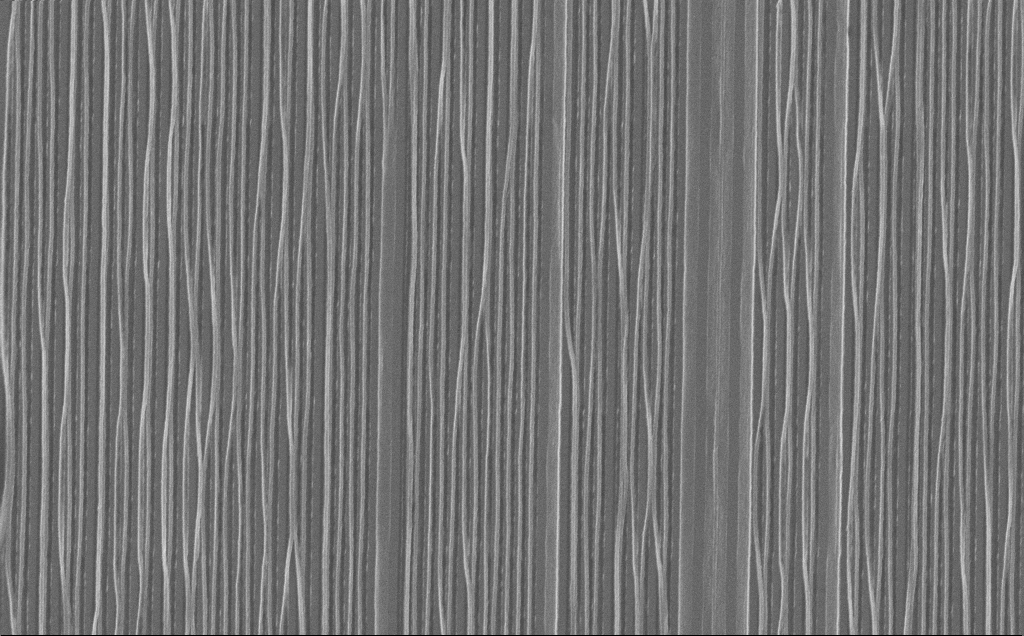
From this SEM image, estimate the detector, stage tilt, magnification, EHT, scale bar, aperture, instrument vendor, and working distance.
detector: InLens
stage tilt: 0°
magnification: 20.69 K X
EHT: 10 kV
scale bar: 2000 nm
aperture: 30 µm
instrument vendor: Zeiss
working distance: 7 mm